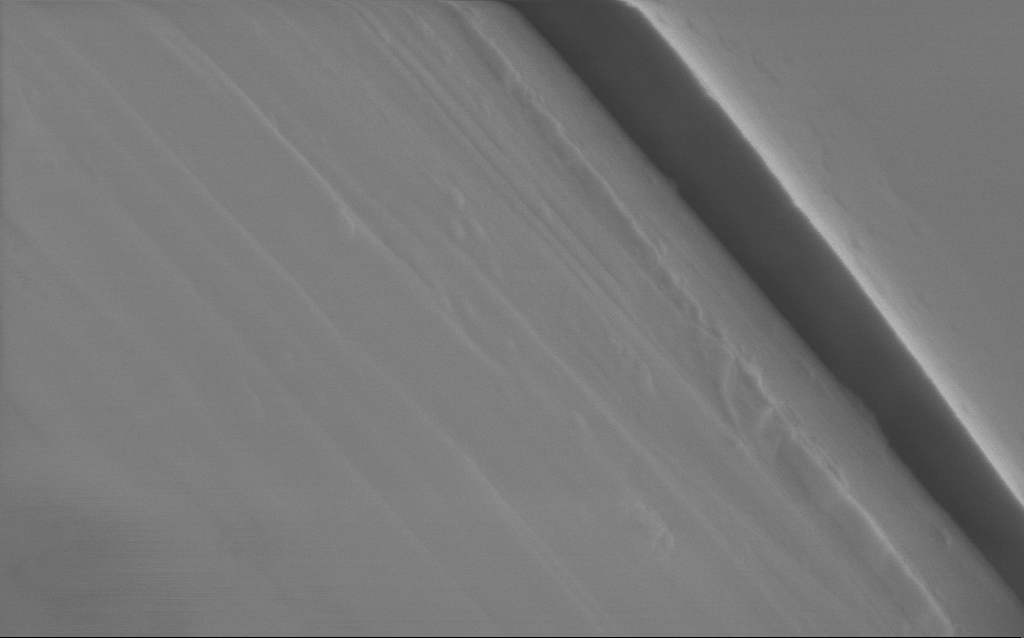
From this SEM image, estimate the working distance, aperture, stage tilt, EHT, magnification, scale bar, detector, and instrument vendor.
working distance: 4 mm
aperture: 30 µm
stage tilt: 0°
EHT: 1 kV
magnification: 39.36 K X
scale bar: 1000 nm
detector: InLens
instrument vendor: Zeiss